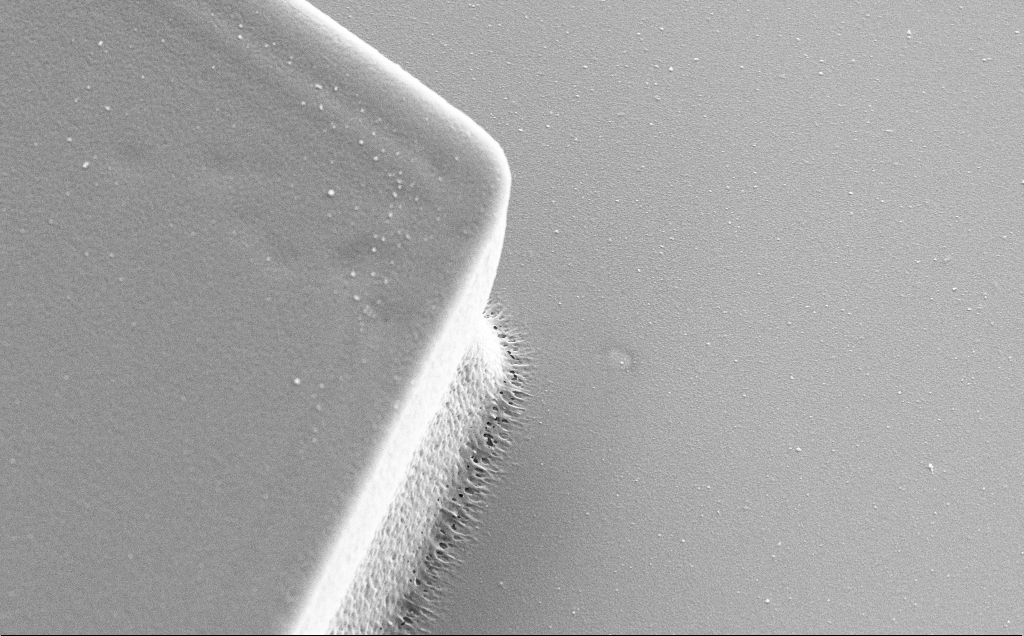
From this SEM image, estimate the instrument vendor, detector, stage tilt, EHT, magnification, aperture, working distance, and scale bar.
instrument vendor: Zeiss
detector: SE2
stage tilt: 30°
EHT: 5 kV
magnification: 14.93 K X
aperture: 30 µm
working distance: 11 mm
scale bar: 1000 nm